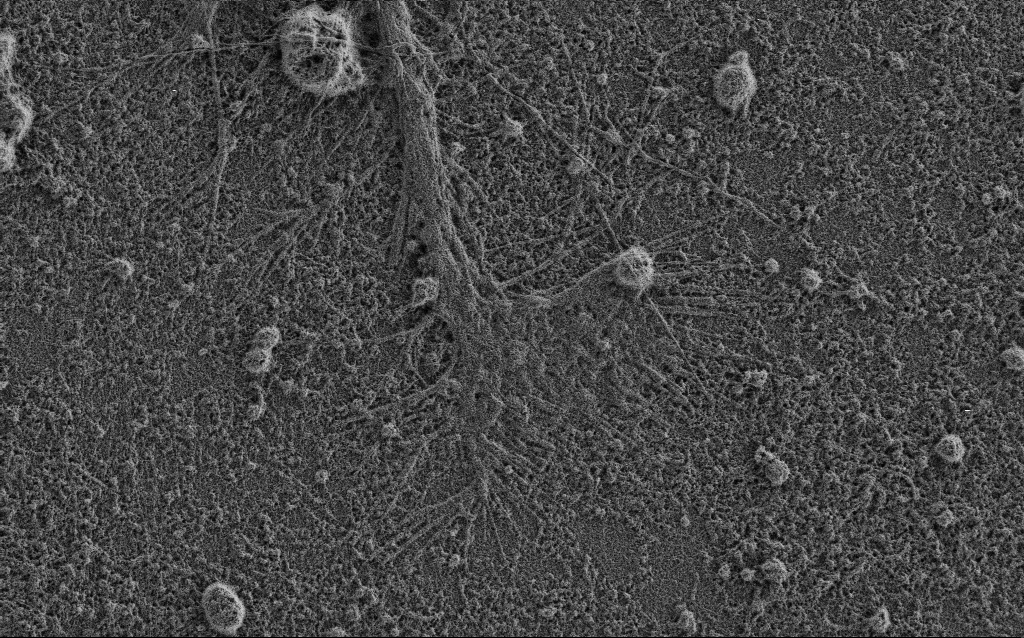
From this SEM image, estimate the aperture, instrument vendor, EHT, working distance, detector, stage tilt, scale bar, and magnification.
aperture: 30 µm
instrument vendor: Zeiss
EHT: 0.9 kV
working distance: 3.4 mm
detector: SE2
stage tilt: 0°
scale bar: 2000 nm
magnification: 7.5 K X